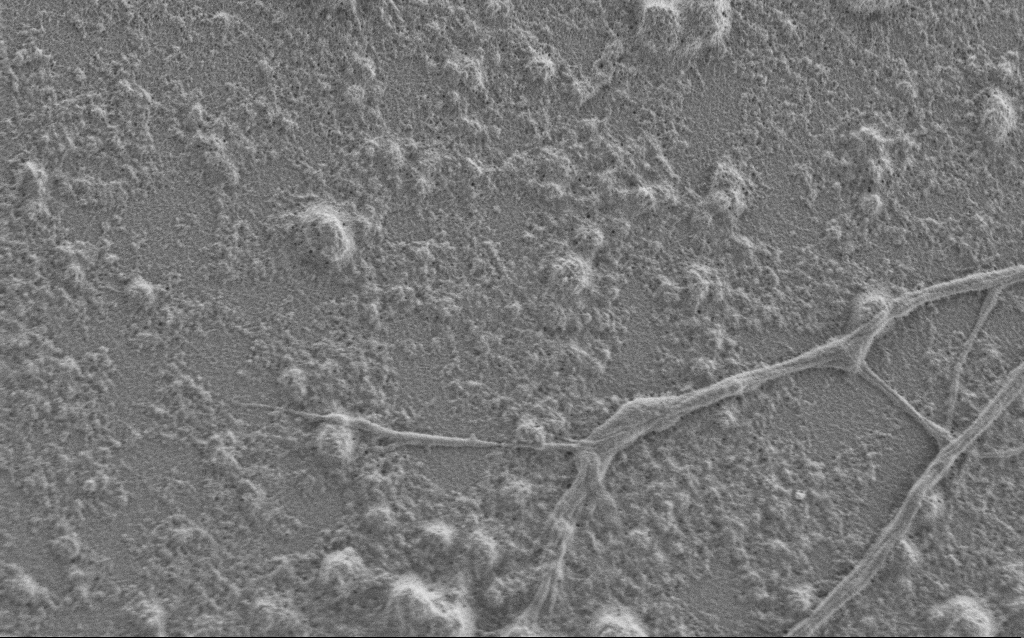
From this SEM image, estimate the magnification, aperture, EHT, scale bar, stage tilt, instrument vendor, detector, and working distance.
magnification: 7.5 K X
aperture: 30 µm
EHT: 1 kV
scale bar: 2000 nm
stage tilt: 0°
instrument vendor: Zeiss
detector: SE2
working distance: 6 mm